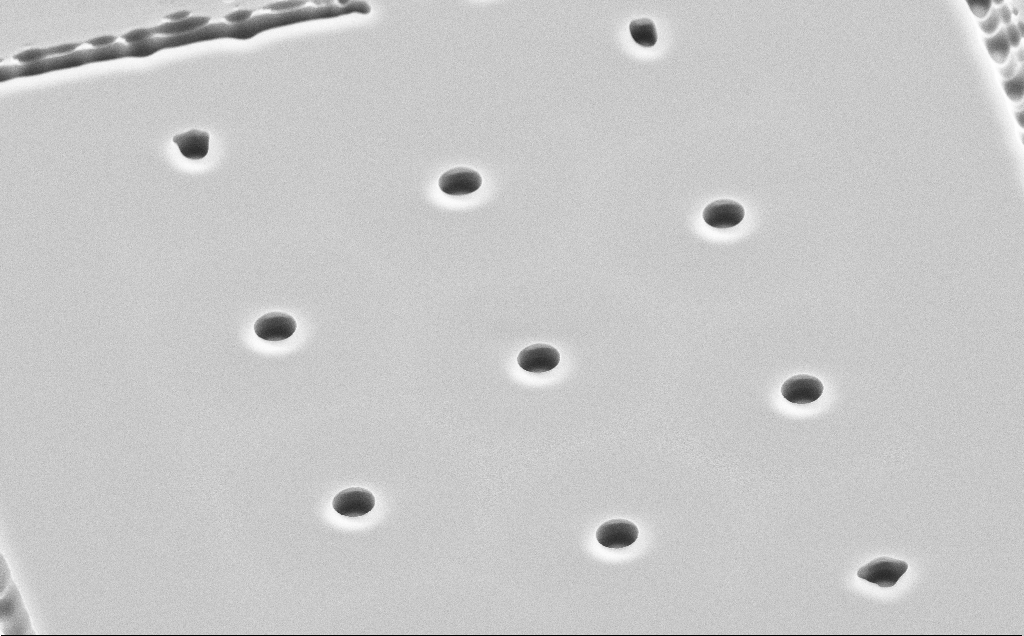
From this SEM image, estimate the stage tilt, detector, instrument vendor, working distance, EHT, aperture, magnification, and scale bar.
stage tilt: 45°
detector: SE2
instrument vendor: Zeiss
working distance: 11 mm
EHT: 10 kV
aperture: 30 µm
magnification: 4.89 K X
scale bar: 10000 nm